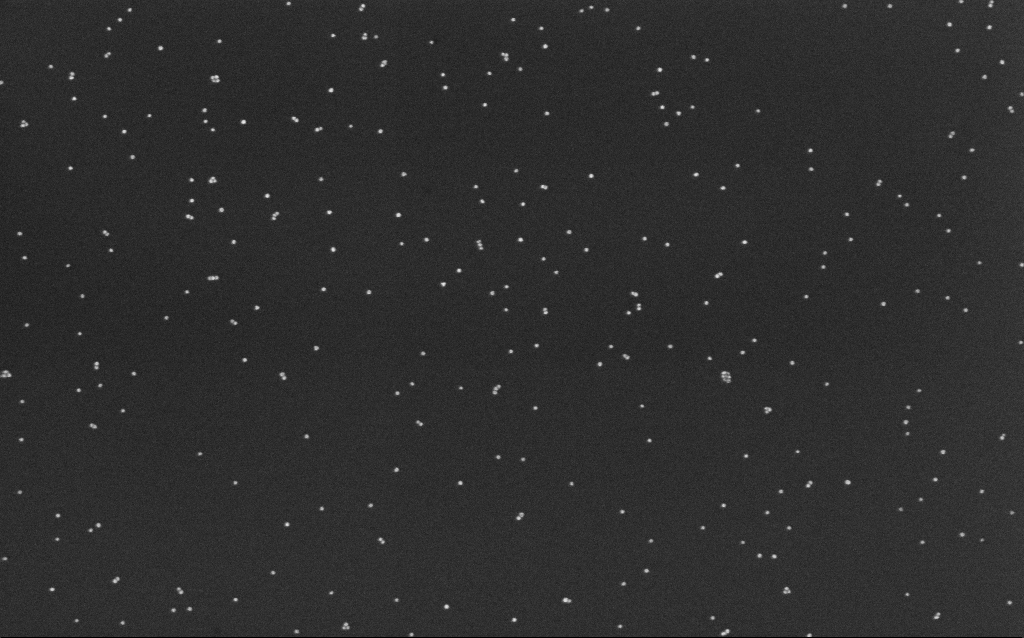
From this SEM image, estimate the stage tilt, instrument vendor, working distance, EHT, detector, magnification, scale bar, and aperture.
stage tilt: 0°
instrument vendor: Zeiss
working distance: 6.6 mm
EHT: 10 kV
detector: InLens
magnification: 100 K X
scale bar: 200 nm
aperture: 30 µm